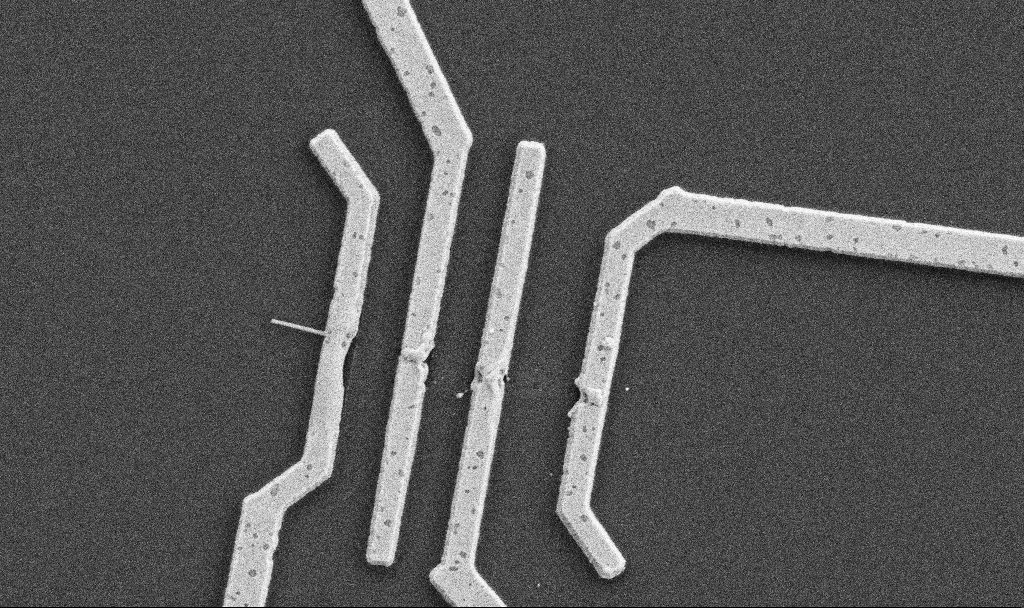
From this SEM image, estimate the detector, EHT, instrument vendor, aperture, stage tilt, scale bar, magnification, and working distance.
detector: SE2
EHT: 5 kV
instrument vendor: Zeiss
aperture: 30 µm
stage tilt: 0°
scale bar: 1000 nm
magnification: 20 K X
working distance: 10.6 mm